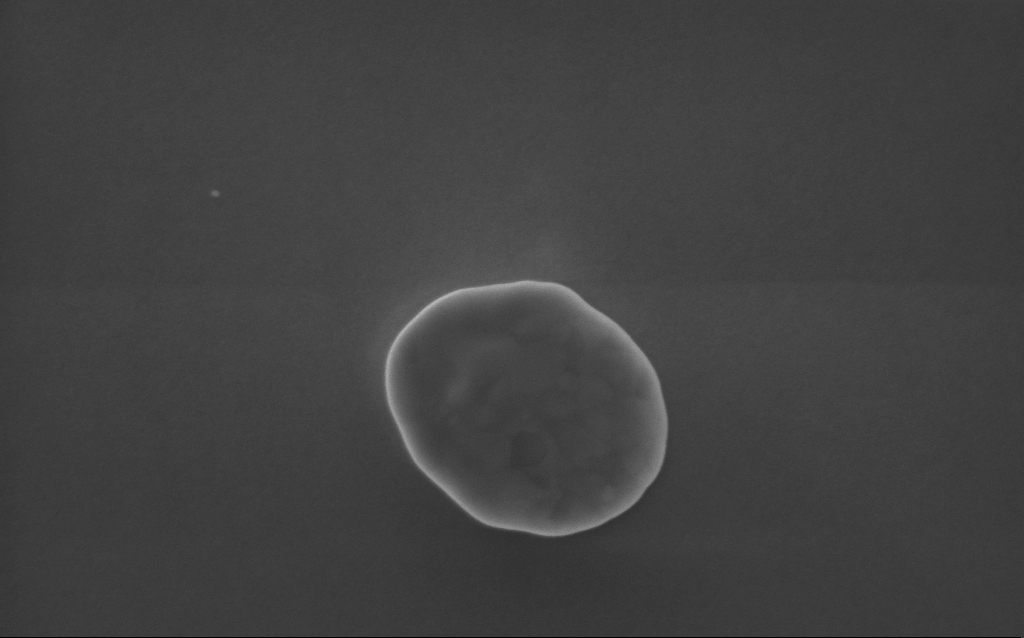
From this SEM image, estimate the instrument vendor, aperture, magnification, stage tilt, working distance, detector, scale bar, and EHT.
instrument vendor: Zeiss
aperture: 30 µm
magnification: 118 K X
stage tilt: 0°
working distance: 3 mm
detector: InLens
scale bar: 200 nm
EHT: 5 kV